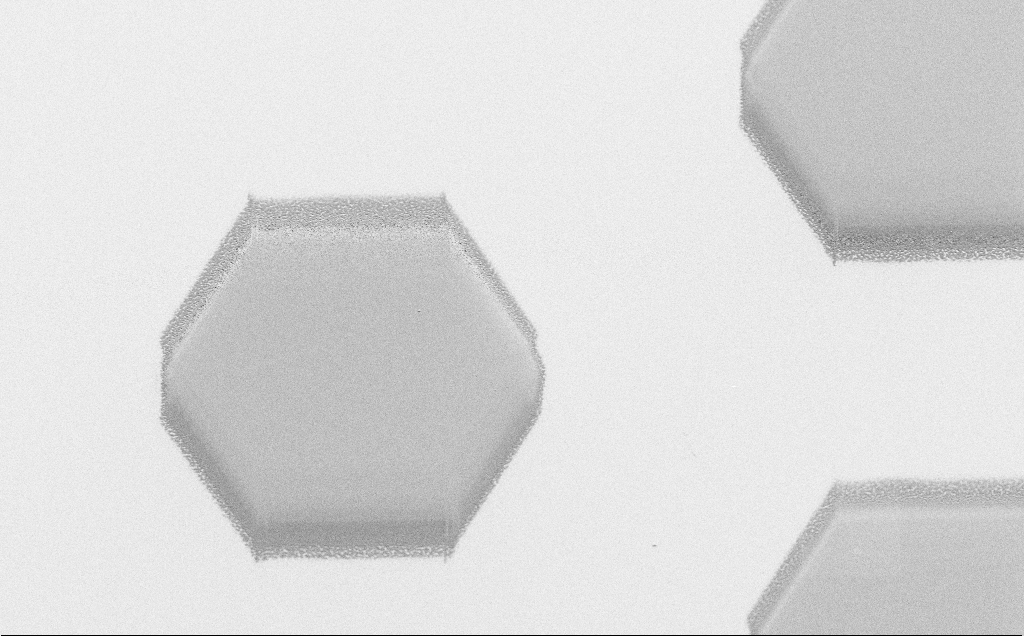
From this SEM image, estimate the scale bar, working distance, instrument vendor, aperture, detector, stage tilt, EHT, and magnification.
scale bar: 20000 nm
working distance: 6 mm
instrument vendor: Zeiss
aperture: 30 µm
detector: SE2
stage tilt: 30°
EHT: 1.5 kV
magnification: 1.23 K X